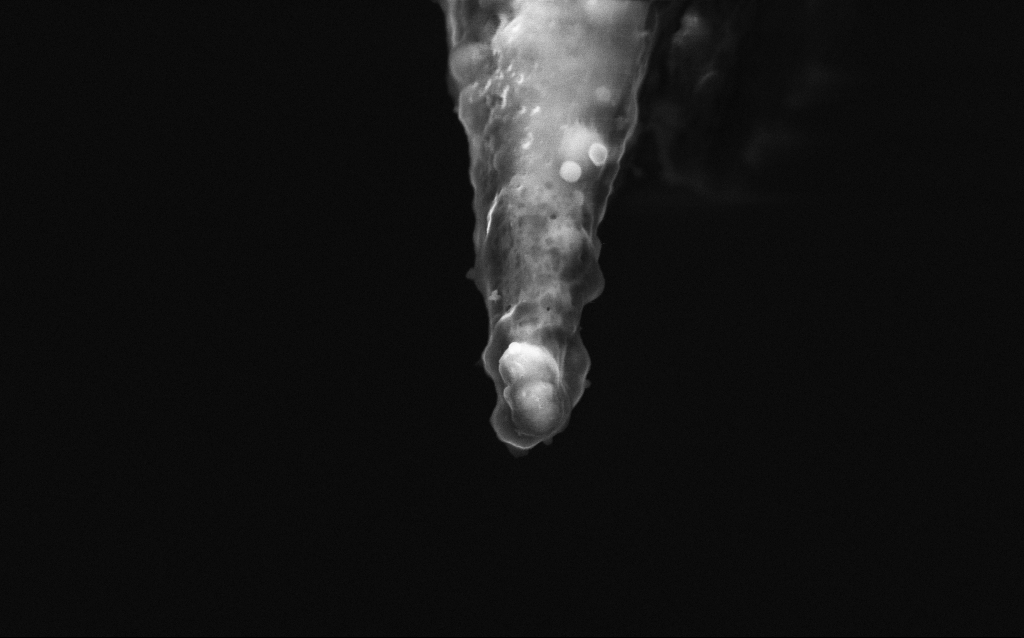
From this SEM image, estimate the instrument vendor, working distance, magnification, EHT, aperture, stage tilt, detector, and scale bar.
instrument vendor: Zeiss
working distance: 6 mm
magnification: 50 K X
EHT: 5 kV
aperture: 30 µm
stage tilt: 43.9°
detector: InLens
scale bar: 1000 nm